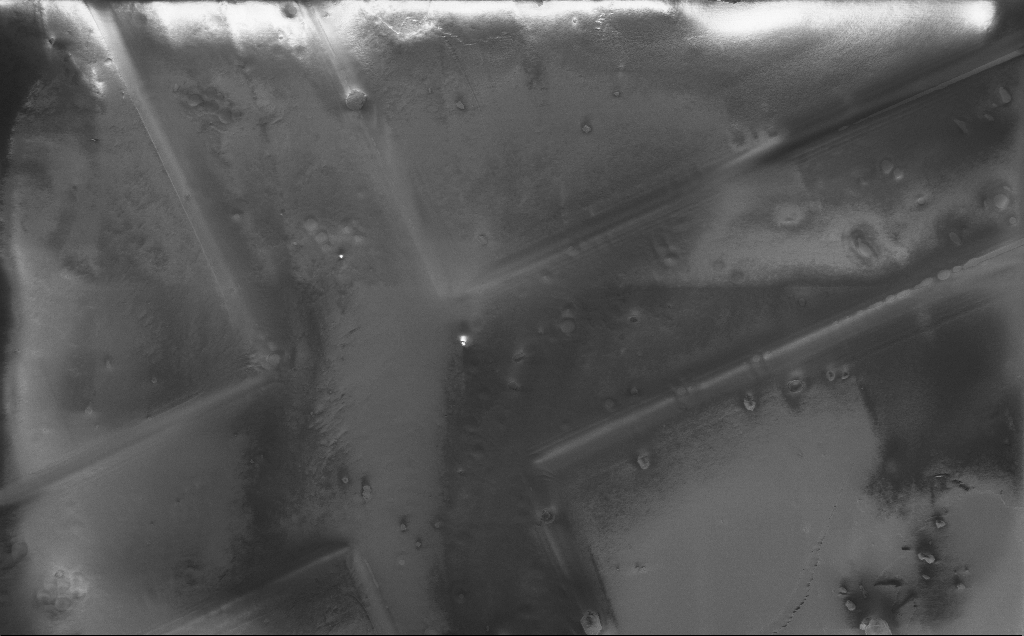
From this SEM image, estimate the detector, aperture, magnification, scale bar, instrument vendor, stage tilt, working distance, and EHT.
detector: InLens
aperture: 30 µm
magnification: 0.729 K X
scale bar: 20000 nm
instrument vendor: Zeiss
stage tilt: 0°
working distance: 5 mm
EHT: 1 kV